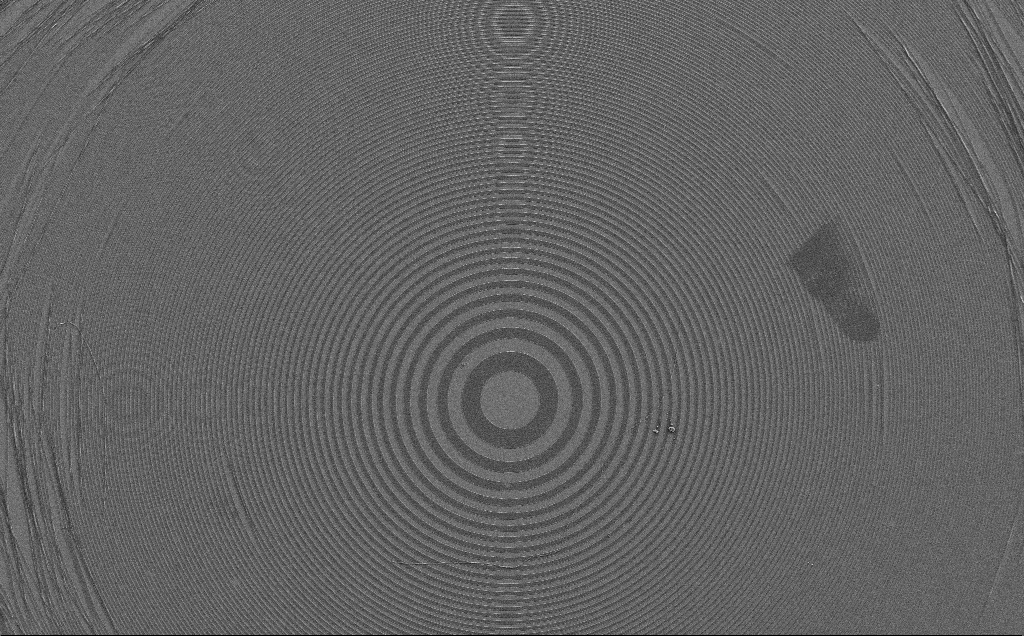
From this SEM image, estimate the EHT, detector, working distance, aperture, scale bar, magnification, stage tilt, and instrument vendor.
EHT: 5 kV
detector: SE2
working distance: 7 mm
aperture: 30 µm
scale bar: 100000 nm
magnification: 0.722 K X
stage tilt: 0°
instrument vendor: Zeiss